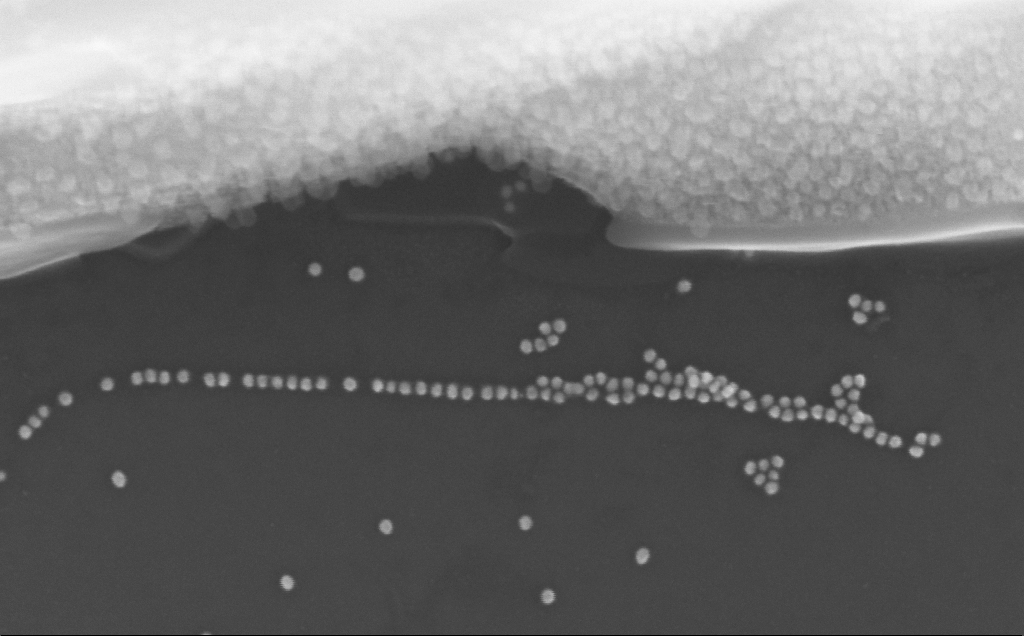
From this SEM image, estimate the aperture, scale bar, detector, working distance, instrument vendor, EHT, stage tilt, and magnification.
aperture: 30 µm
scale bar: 200 nm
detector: InLens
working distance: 3 mm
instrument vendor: Zeiss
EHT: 10 kV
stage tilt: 0°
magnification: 284.35 K X